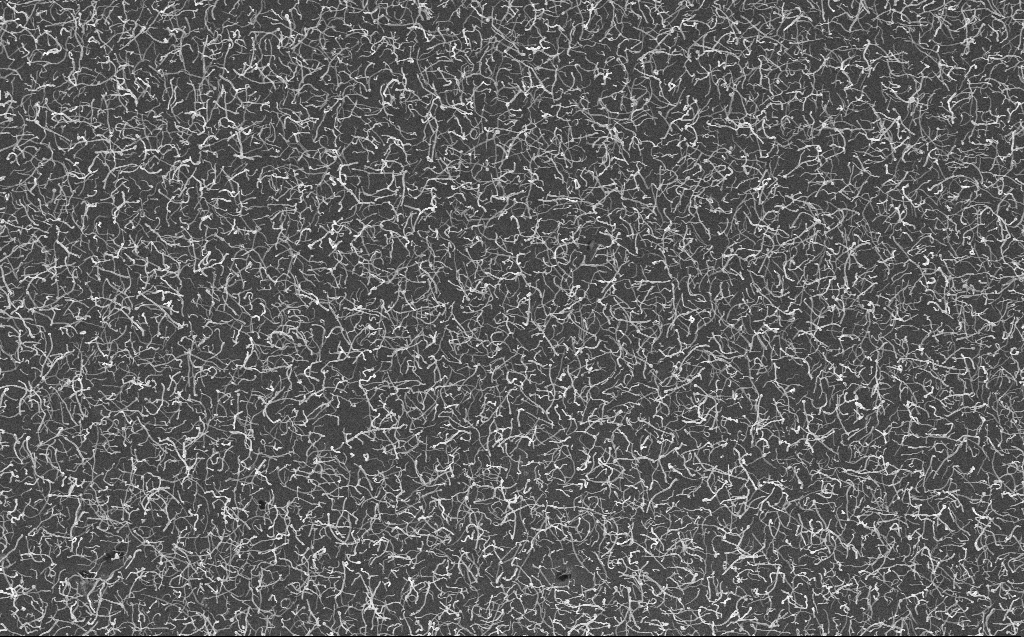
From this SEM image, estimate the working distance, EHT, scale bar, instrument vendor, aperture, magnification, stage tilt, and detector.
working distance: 3 mm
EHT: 10 kV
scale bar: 2000 nm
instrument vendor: Zeiss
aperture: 30 µm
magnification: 10 K X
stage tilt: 0°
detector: InLens